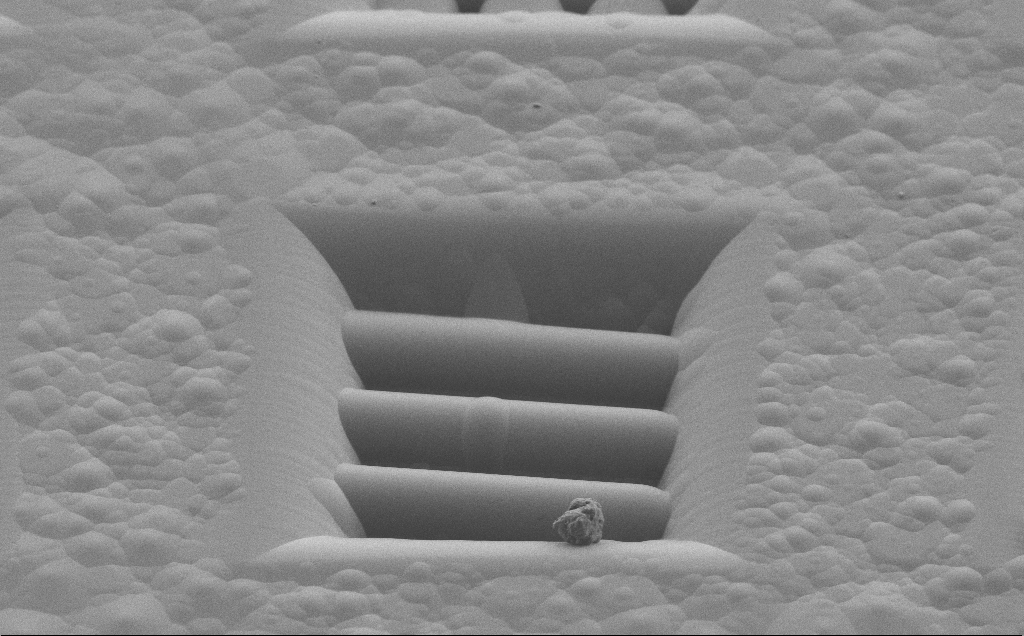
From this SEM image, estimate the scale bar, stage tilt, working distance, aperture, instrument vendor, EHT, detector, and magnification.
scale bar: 20000 nm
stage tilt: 45°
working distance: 7 mm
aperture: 30 µm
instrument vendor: Zeiss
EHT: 1.3 kV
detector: SE2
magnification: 1.88 K X